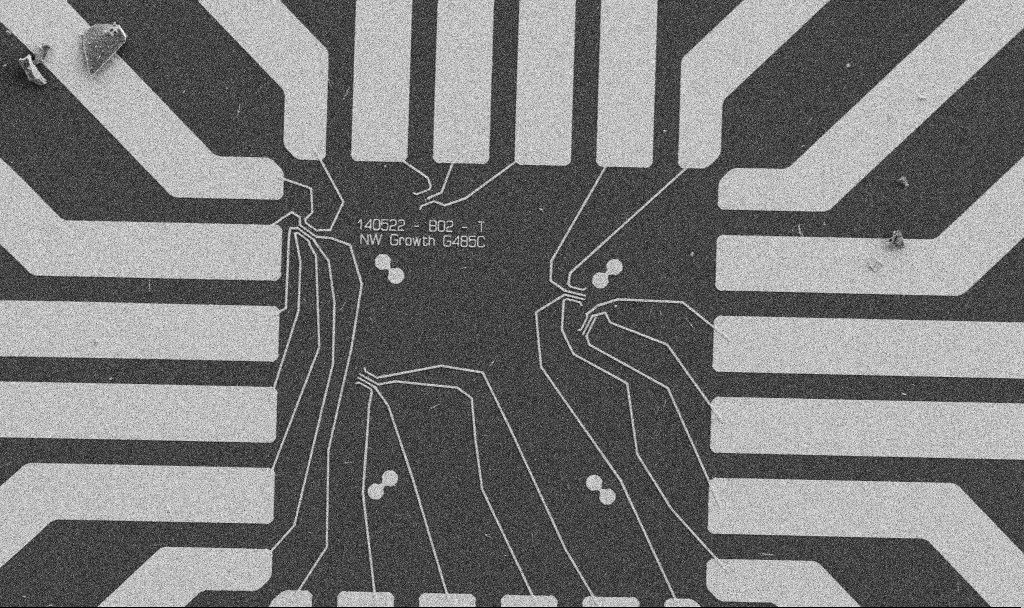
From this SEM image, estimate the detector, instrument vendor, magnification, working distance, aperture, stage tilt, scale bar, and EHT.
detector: SE2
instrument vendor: Zeiss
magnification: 1 K X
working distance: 10.6 mm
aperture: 30 µm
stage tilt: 0°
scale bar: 20000 nm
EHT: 5 kV